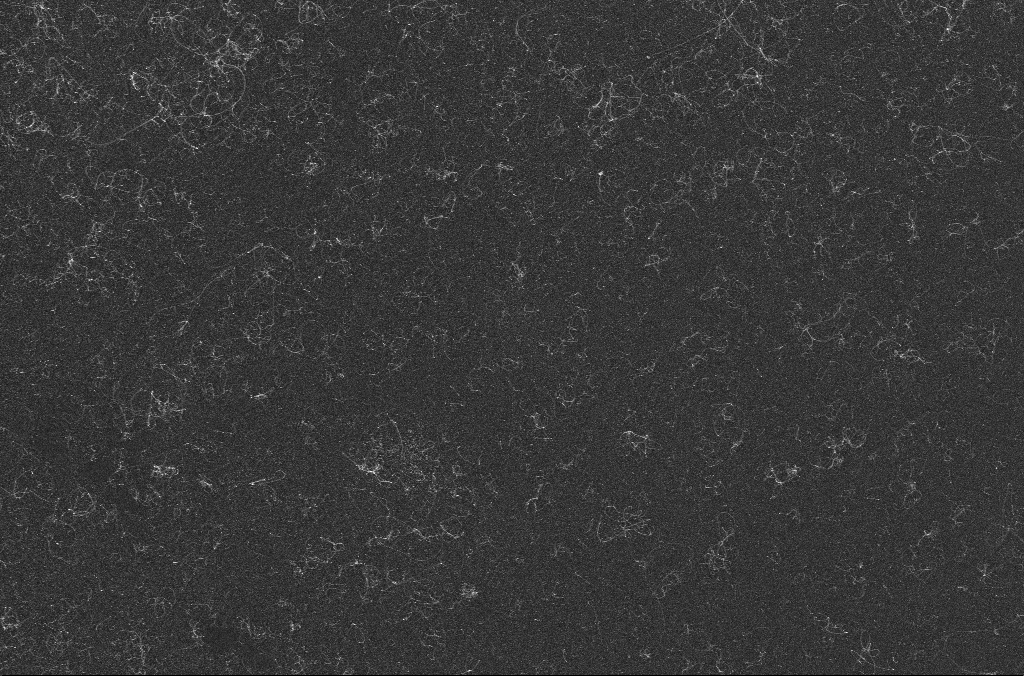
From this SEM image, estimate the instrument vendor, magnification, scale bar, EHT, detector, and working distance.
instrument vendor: Zeiss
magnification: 15 K X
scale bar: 1000 nm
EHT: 10 kV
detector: InLens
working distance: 3.1 mm